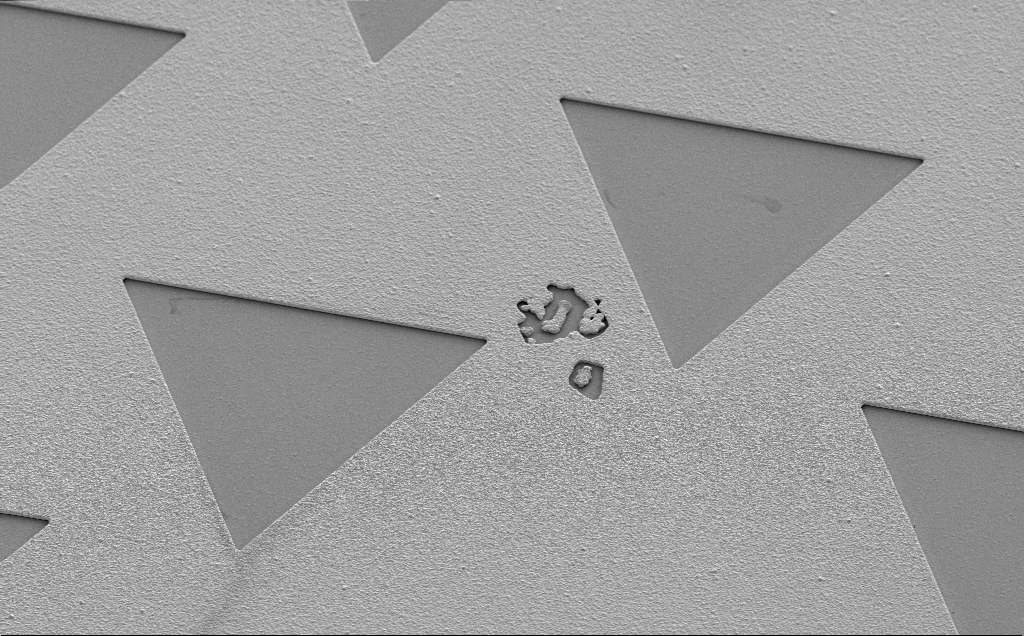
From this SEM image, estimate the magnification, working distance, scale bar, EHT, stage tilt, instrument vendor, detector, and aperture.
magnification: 1.18 K X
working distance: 13 mm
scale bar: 20000 nm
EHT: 5 kV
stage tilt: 35°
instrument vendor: Zeiss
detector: SE2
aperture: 30 µm